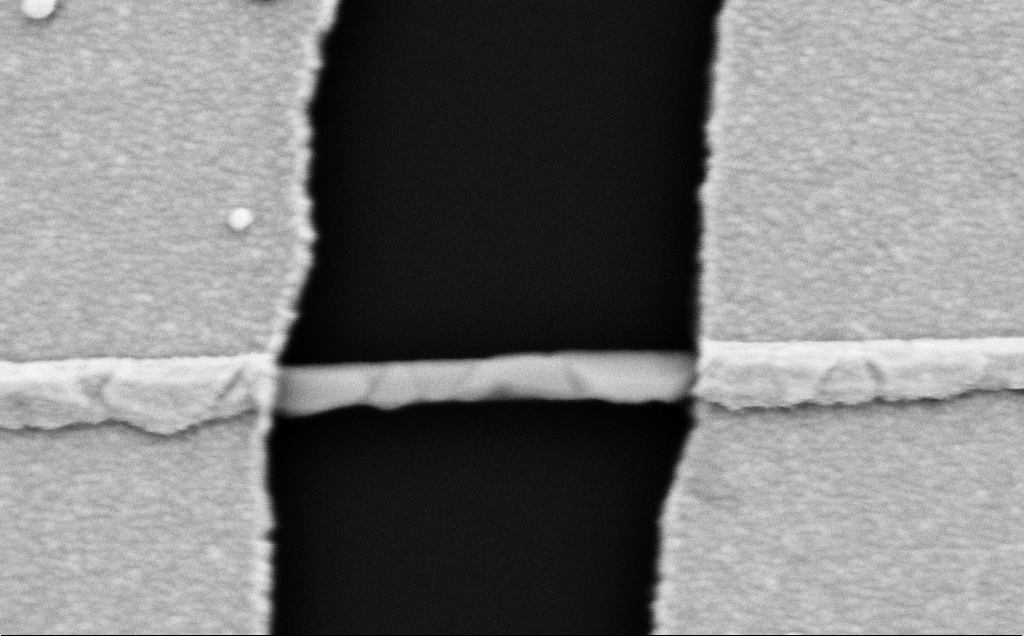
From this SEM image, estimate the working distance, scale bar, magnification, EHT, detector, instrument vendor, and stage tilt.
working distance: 10 mm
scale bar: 200 nm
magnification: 101.77 K X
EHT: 5 kV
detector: SE2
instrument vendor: Zeiss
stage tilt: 0°